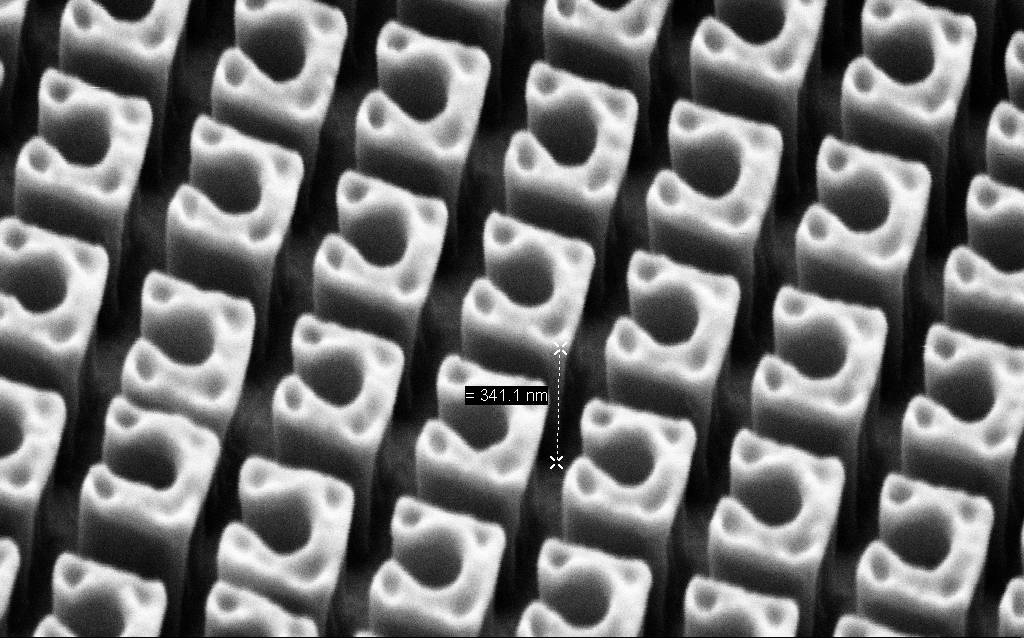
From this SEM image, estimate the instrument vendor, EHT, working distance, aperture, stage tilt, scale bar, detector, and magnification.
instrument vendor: Zeiss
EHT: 3 kV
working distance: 7.2 mm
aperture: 30 µm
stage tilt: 45°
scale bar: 200 nm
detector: SE2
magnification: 122.81 K X